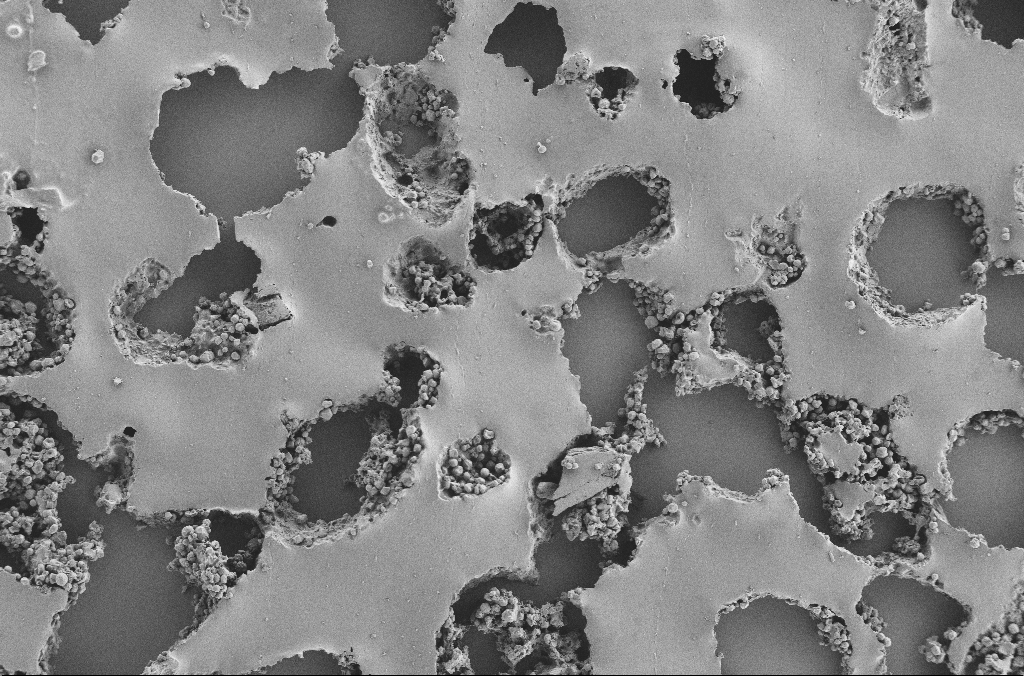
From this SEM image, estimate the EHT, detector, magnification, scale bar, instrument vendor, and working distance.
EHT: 2 kV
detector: SE2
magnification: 0.25 K X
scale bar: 100000 nm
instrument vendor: Zeiss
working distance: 3.7 mm